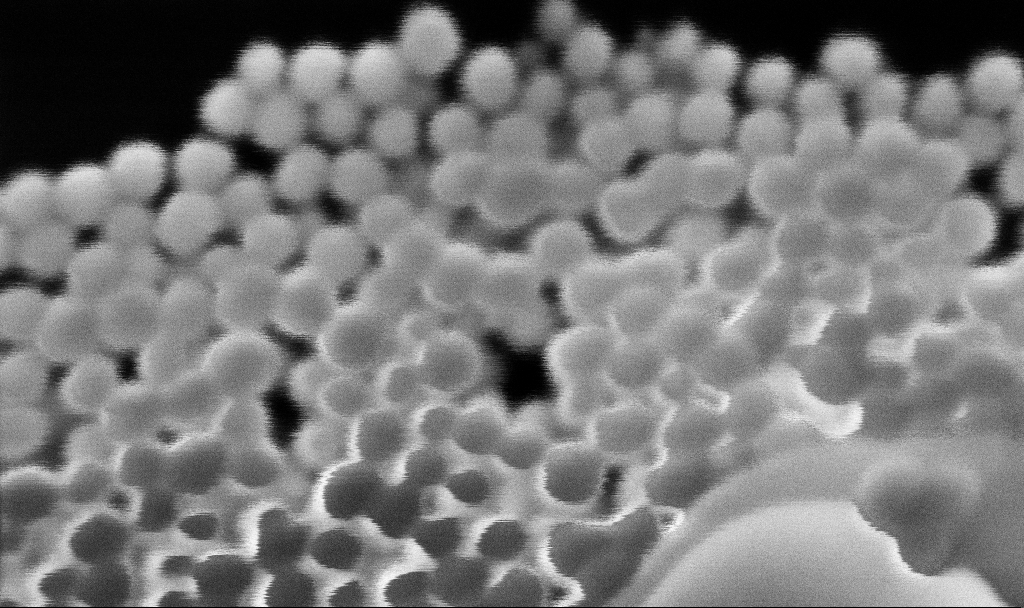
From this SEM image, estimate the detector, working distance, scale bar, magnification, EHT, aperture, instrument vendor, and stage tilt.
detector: InLens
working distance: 3.1 mm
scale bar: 20 nm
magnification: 1096.25 K X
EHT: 4 kV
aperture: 30 µm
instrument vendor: Zeiss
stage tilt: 0°